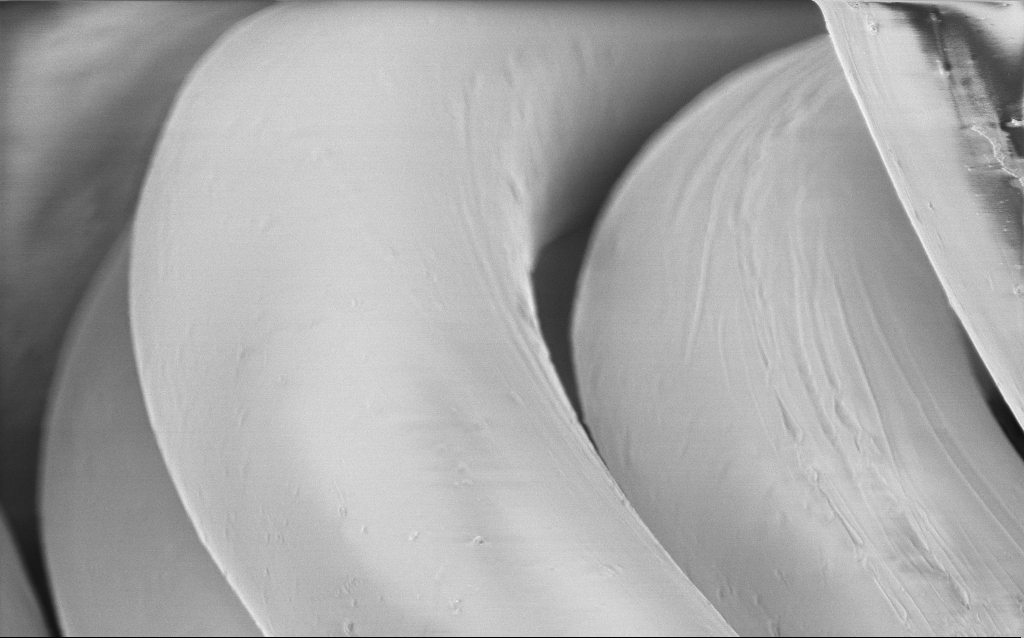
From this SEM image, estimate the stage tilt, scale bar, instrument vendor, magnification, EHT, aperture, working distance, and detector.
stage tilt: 0°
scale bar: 10000 nm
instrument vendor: Zeiss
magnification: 6.24 K X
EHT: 2 kV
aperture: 30 µm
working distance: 5 mm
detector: InLens